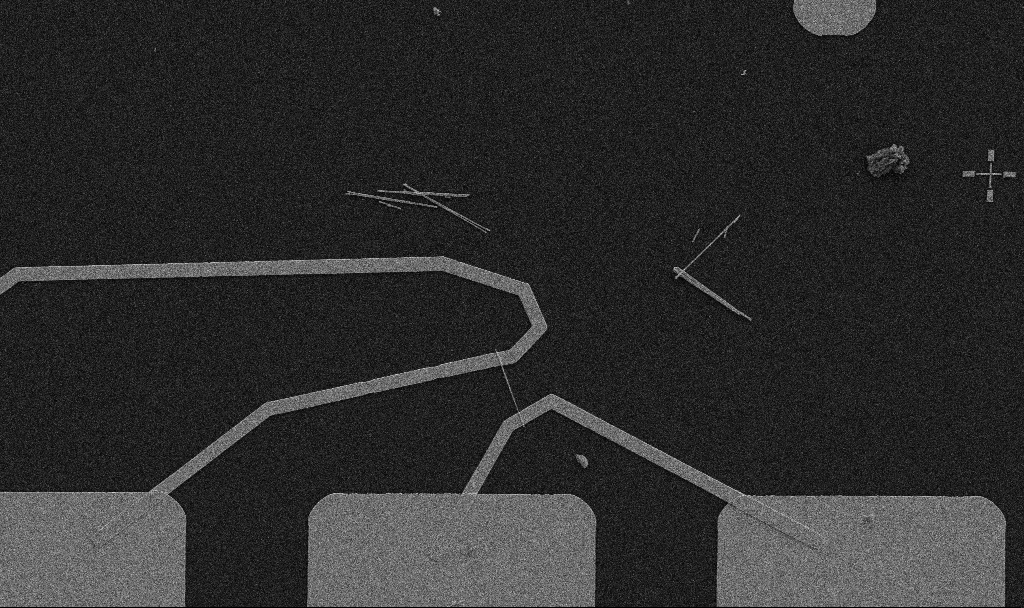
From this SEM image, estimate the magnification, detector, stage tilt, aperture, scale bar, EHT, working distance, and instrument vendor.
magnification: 5 K X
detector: SE2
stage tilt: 0°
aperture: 30 µm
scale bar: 10000 nm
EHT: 5 kV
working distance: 10.7 mm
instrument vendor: Zeiss